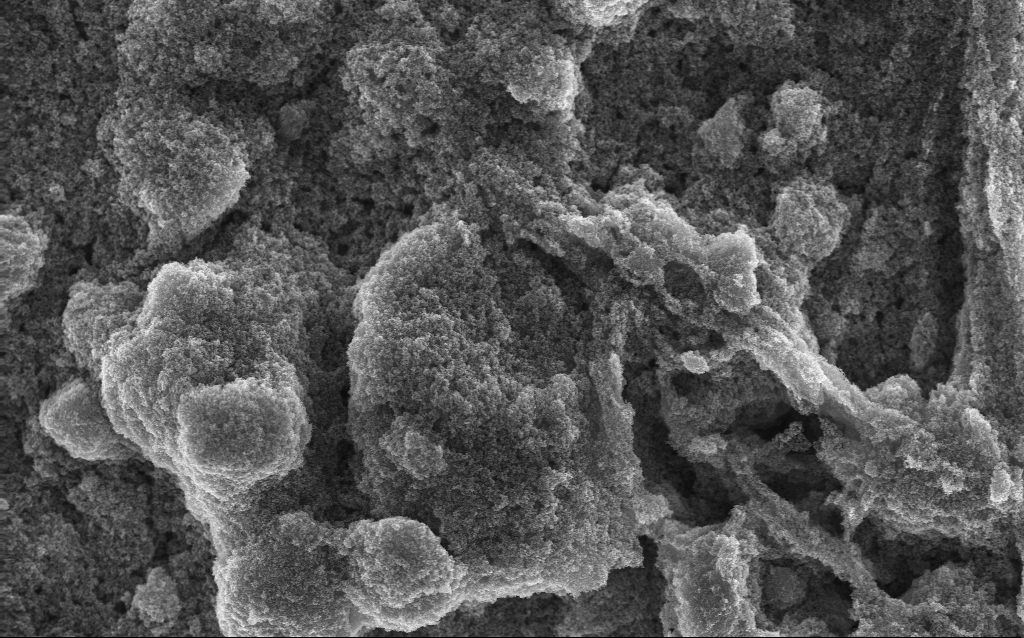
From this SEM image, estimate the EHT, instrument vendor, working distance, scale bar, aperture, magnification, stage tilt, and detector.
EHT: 10 kV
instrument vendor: Zeiss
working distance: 2.8 mm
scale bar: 1000 nm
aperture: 30 µm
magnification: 15.33 K X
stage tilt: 0°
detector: InLens